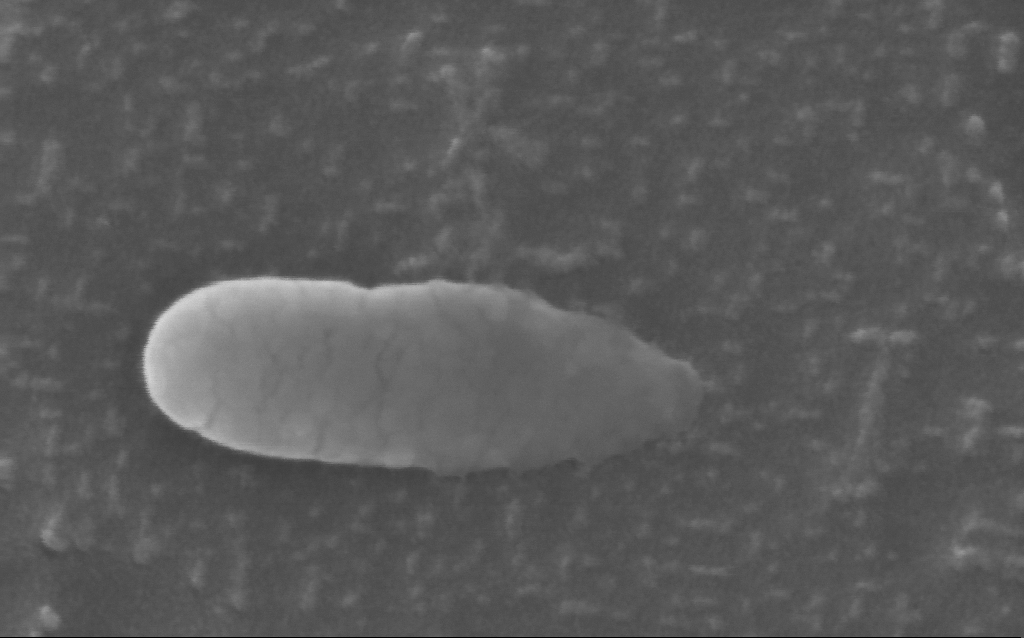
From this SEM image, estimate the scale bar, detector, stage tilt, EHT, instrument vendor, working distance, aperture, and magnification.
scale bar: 100 nm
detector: InLens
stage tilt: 21.3°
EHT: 10 kV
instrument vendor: Zeiss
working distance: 6 mm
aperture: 30 µm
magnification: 533.19 K X